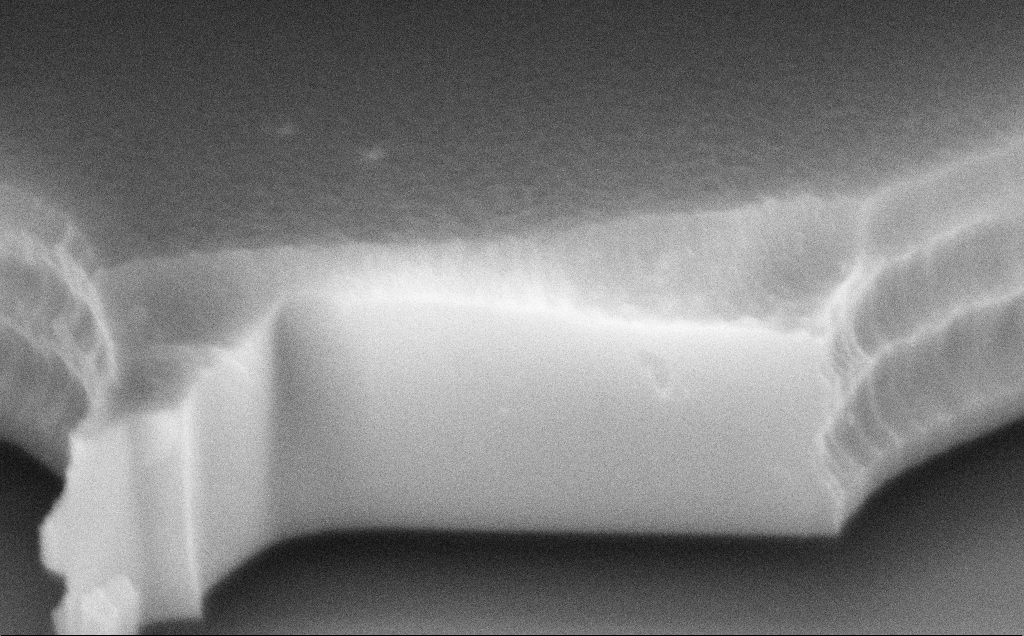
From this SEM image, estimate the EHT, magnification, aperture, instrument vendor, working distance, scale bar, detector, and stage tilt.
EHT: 8 kV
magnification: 52 K X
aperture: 30 µm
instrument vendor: Zeiss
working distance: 12 mm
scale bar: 1000 nm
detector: InLens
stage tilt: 70°